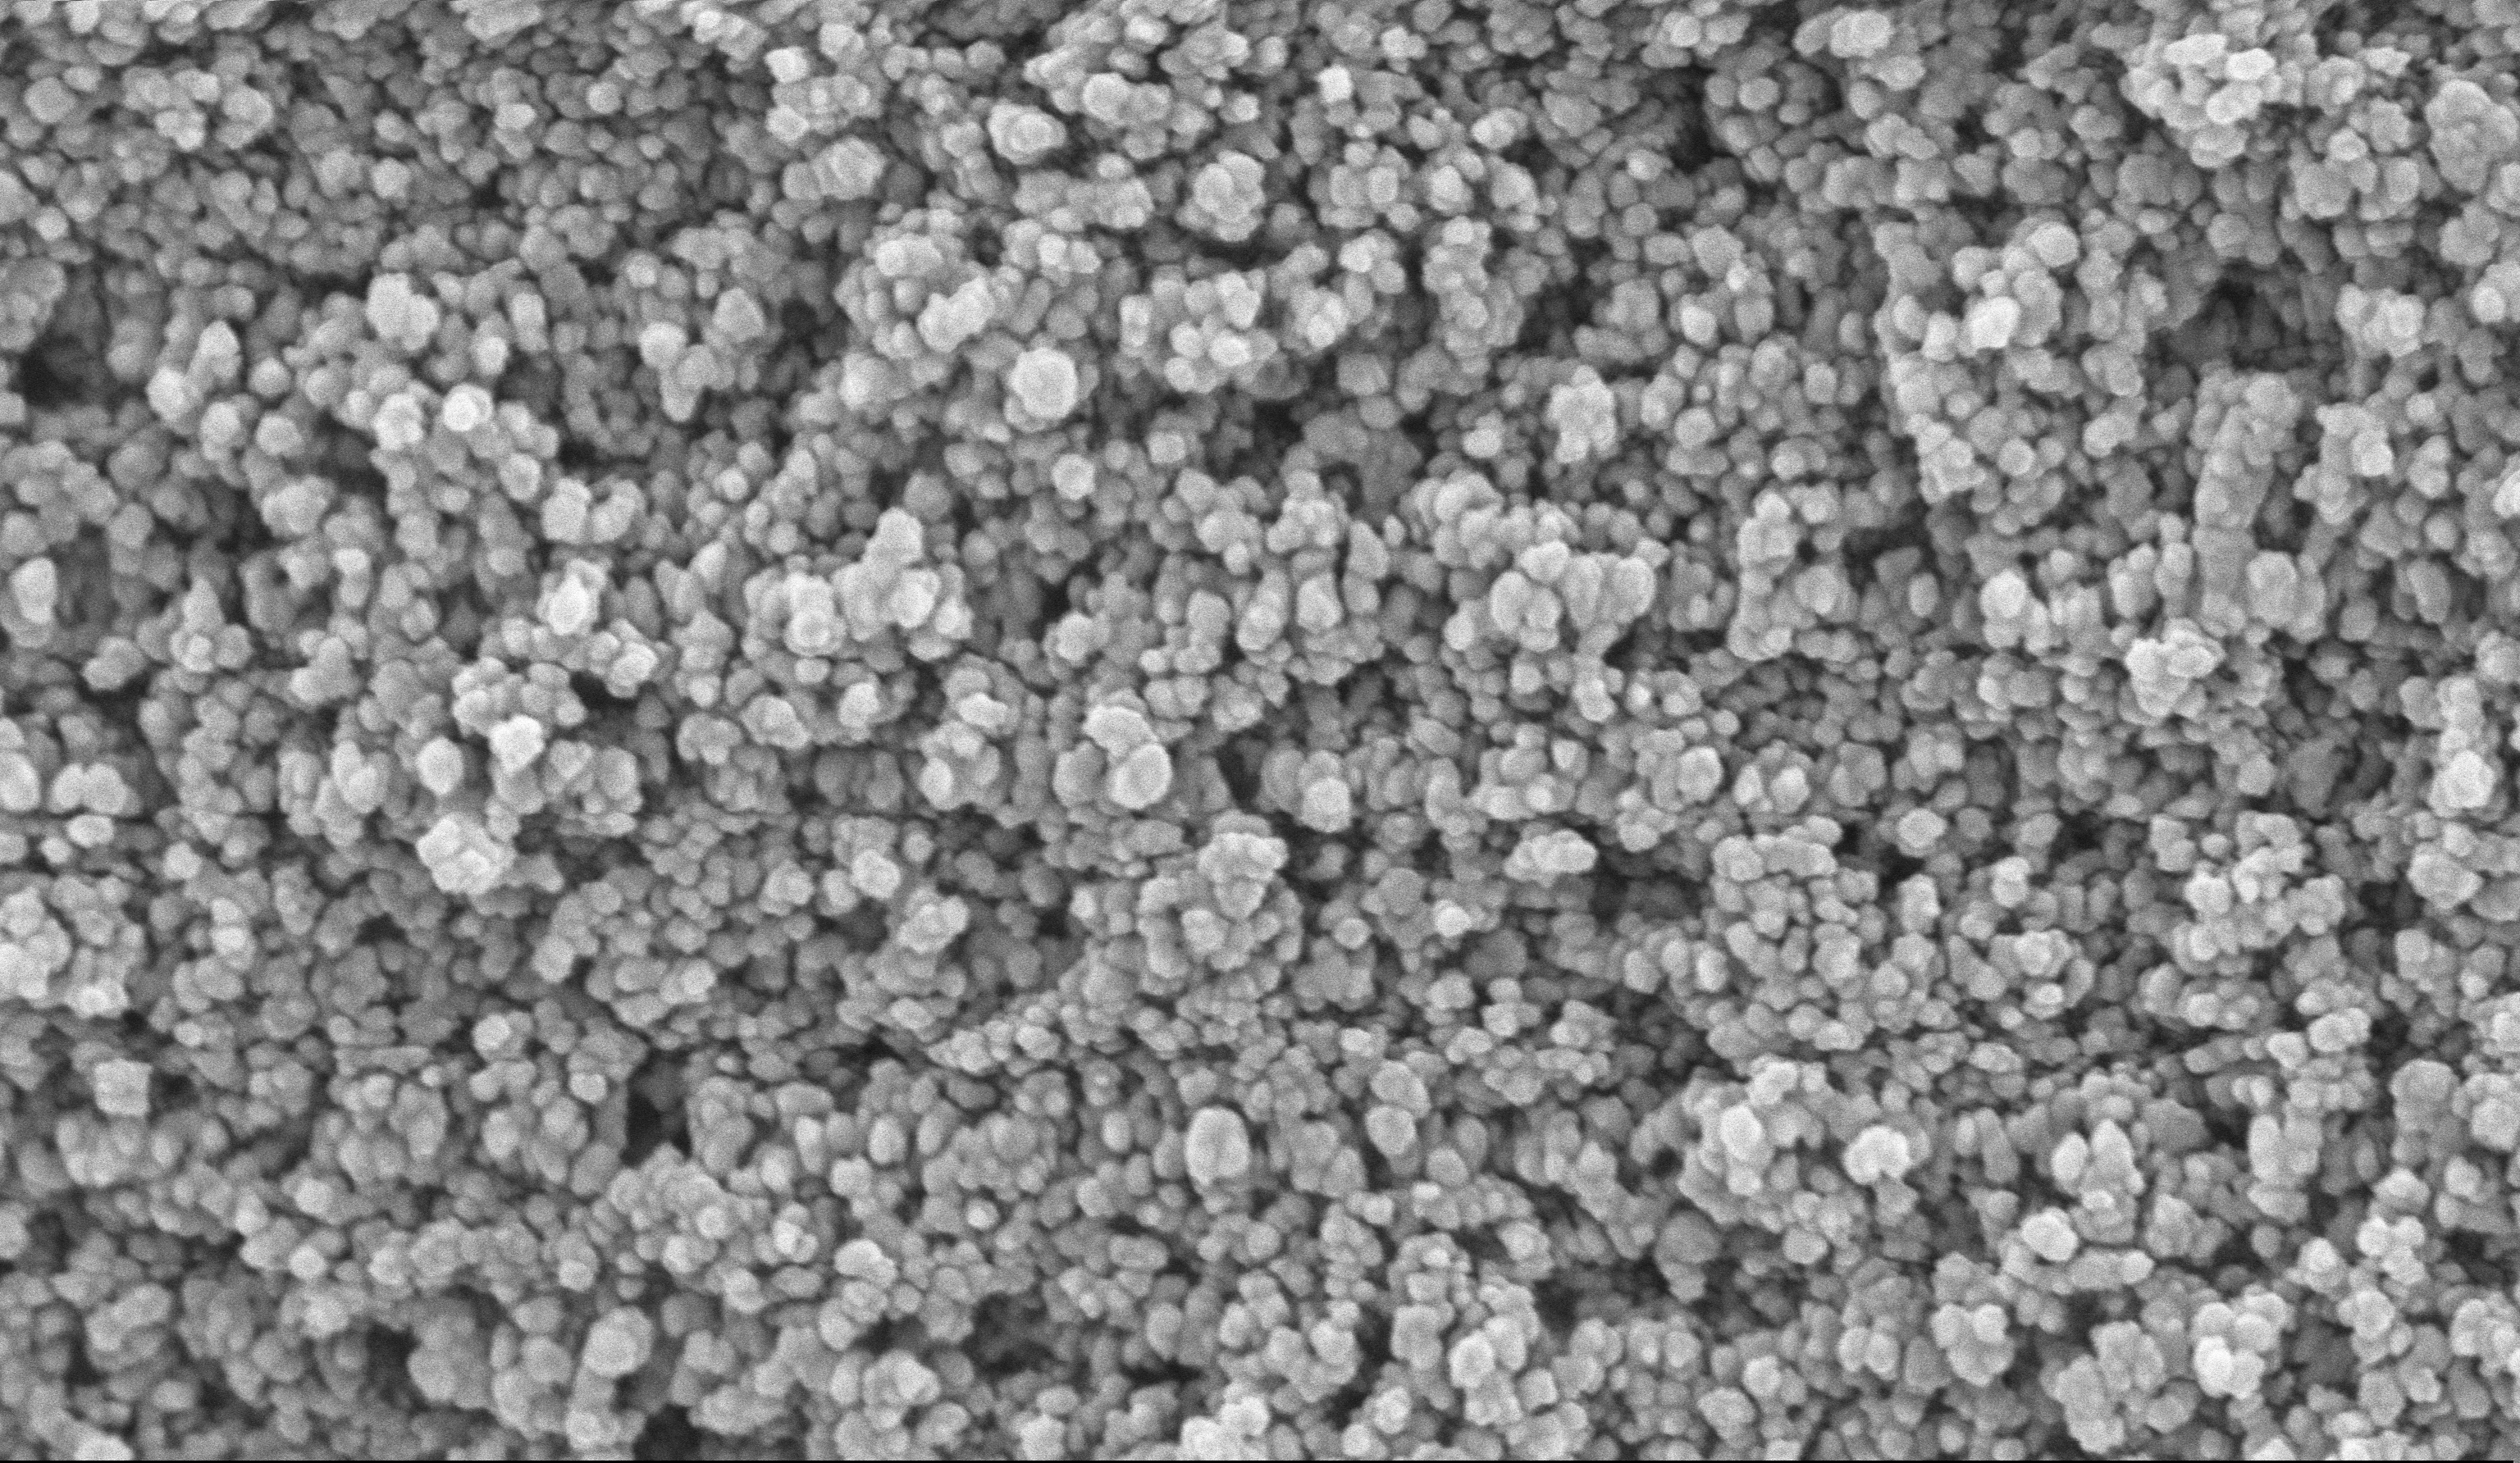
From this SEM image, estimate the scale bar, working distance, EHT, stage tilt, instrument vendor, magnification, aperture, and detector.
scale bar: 100 nm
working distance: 5.1 mm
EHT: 10 kV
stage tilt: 0°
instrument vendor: Zeiss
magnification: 135 K X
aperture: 30 µm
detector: InLens